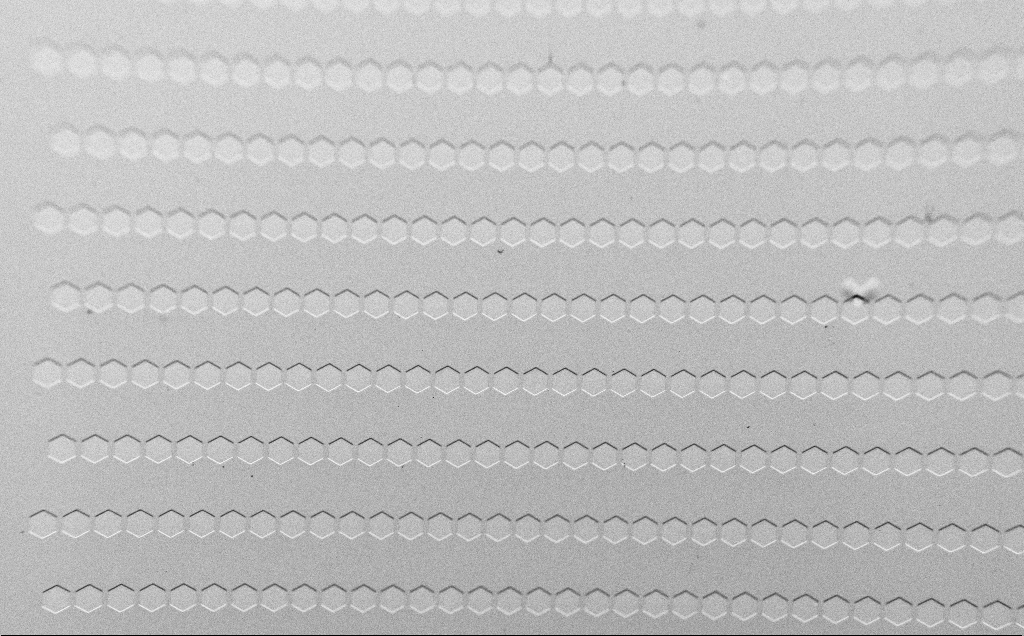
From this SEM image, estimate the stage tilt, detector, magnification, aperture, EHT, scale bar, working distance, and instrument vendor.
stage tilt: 45°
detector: SE2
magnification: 0.186 K X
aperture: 30 µm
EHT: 1.5 kV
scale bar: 200000 nm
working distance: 4 mm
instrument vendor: Zeiss